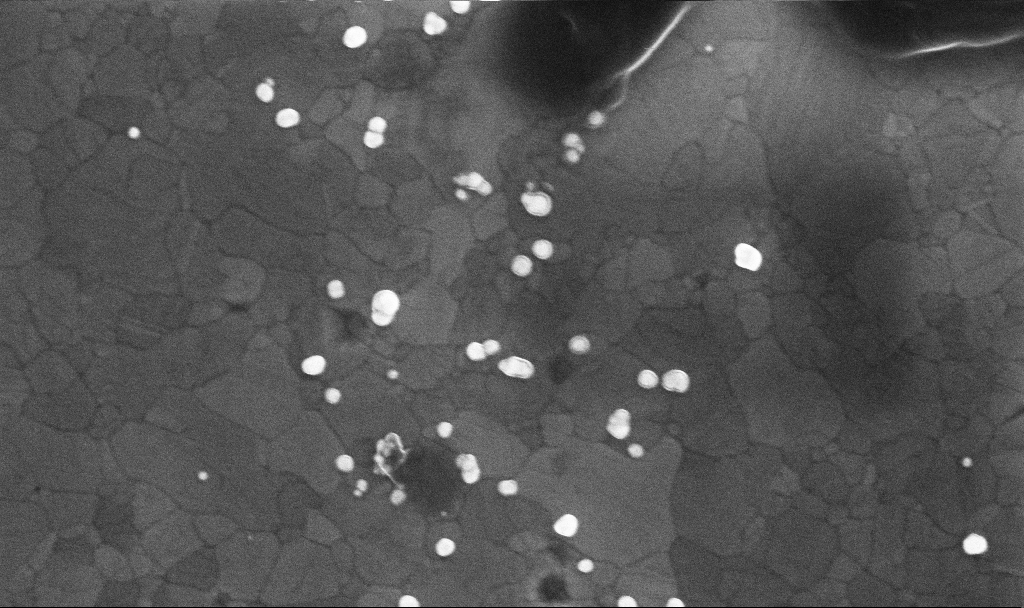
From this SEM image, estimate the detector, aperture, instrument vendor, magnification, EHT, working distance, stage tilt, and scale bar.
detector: InLens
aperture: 30 µm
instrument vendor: Zeiss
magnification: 117.41 K X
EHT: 10 kV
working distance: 3.4 mm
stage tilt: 0°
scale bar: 200 nm